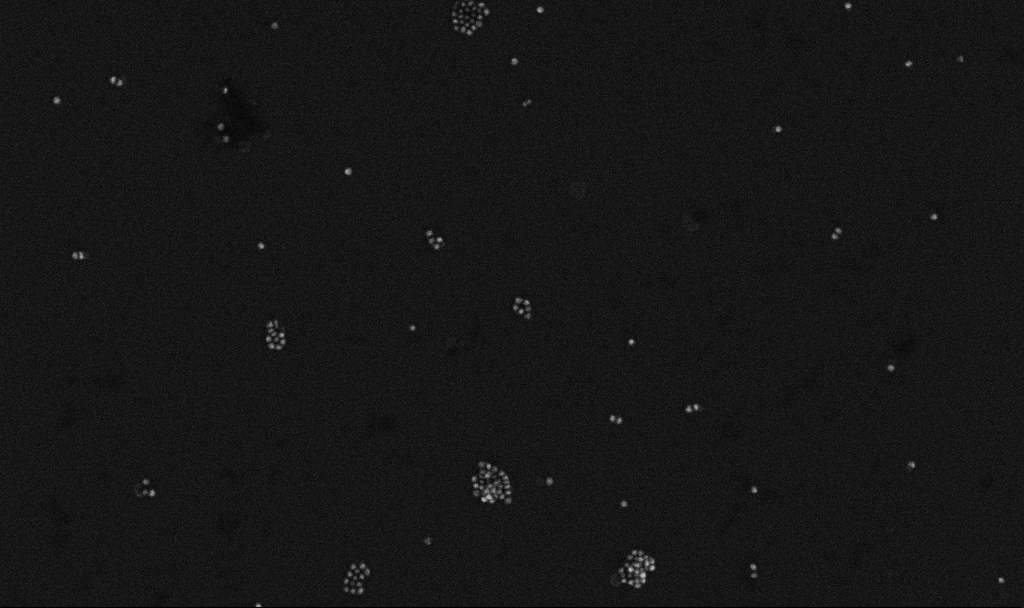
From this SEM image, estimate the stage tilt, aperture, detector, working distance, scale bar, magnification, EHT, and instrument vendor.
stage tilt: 0°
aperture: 30 µm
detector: InLens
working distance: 3.3 mm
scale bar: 200 nm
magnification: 100 K X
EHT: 10 kV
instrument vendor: Zeiss